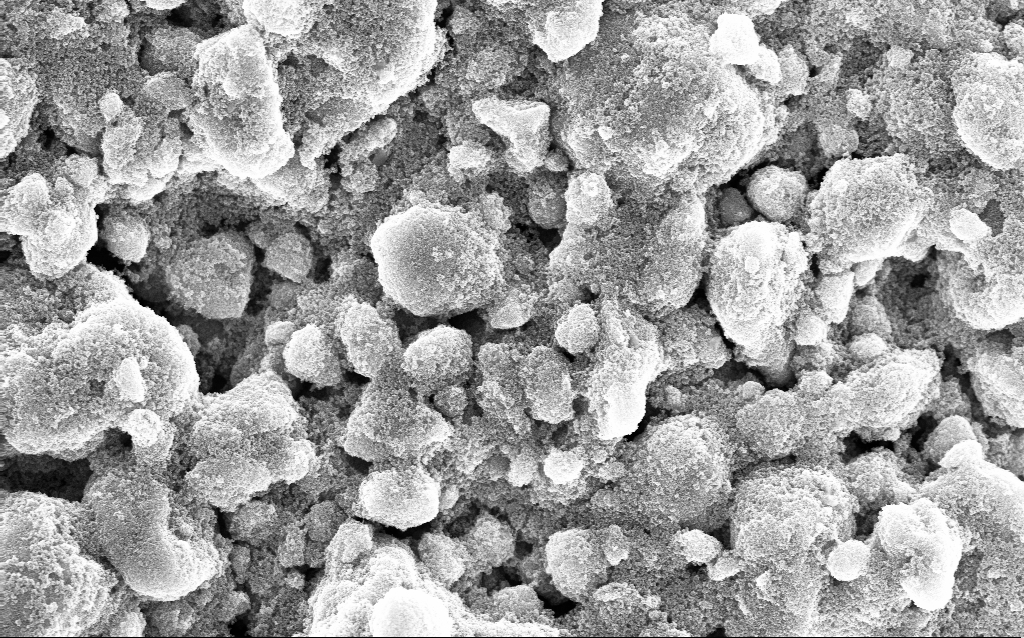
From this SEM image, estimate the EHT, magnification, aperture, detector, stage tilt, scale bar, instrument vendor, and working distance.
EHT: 10 kV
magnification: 14.34 K X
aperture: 30 µm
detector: InLens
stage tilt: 0°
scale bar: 2000 nm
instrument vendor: Zeiss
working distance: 3.8 mm